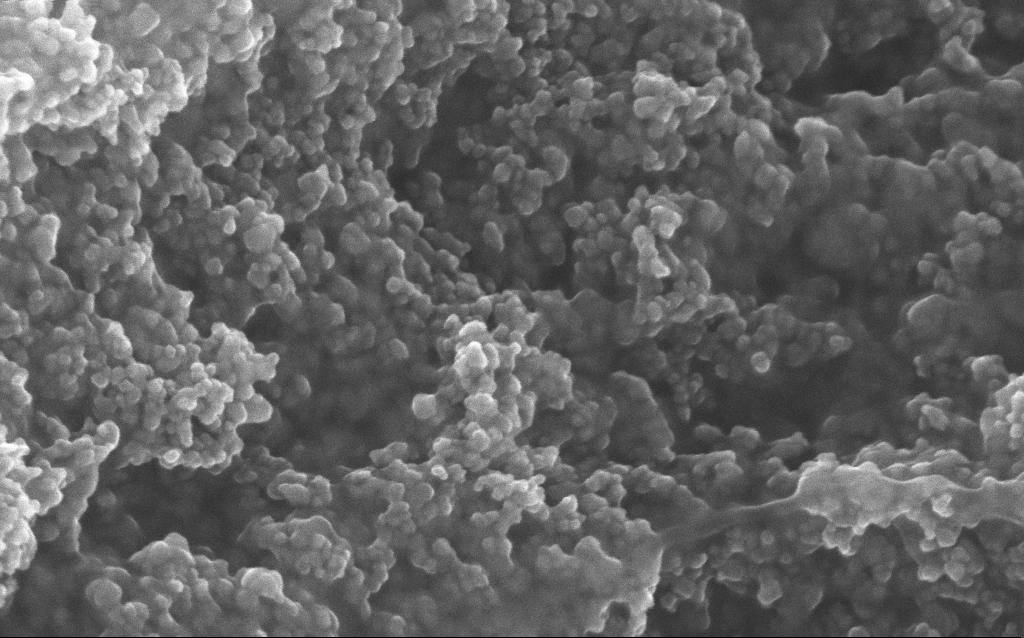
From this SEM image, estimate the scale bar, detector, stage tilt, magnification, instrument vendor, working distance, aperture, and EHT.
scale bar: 100 nm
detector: InLens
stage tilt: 0°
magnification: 211.33 K X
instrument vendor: Zeiss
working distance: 2.8 mm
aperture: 30 µm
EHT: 10 kV